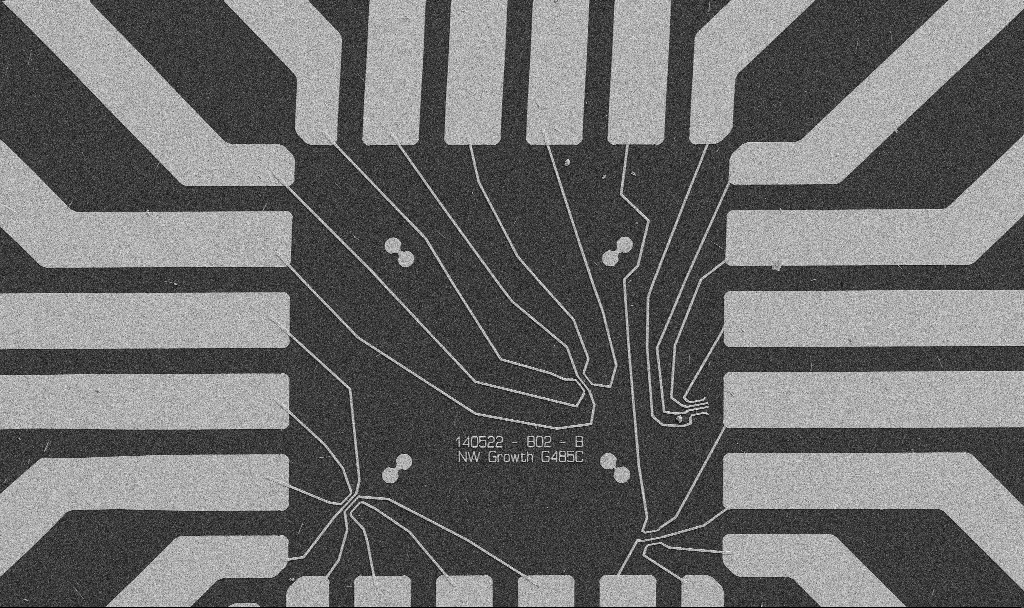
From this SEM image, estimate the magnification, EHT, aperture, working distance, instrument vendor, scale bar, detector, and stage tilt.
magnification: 1 K X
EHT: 5 kV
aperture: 30 µm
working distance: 10.7 mm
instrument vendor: Zeiss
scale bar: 20000 nm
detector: SE2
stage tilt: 0°